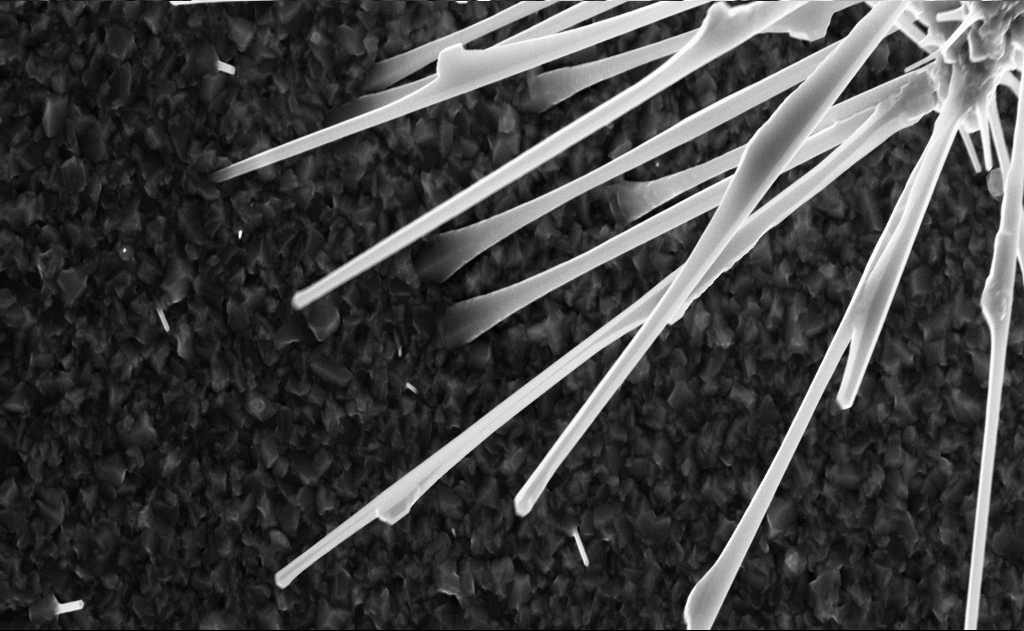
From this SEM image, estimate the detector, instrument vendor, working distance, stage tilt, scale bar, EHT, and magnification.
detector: InLens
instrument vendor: Zeiss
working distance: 6 mm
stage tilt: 0°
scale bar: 1000 nm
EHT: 10 kV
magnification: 20 K X